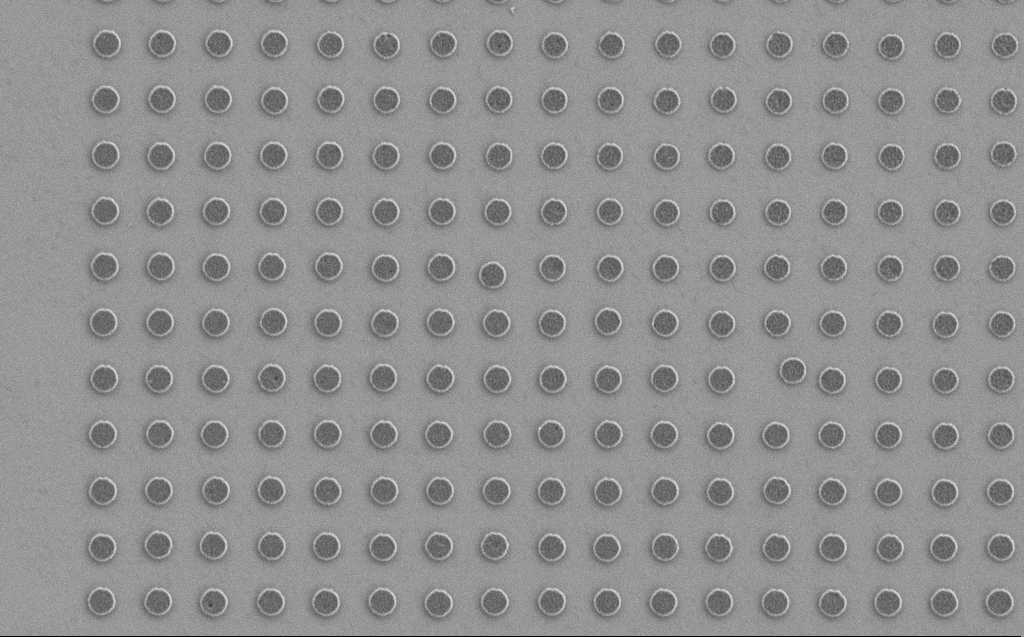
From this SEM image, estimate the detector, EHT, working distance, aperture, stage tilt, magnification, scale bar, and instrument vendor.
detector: SE2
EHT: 1.2 kV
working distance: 5 mm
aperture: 30 µm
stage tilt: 0°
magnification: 17.19 K X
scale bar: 2000 nm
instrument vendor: Zeiss